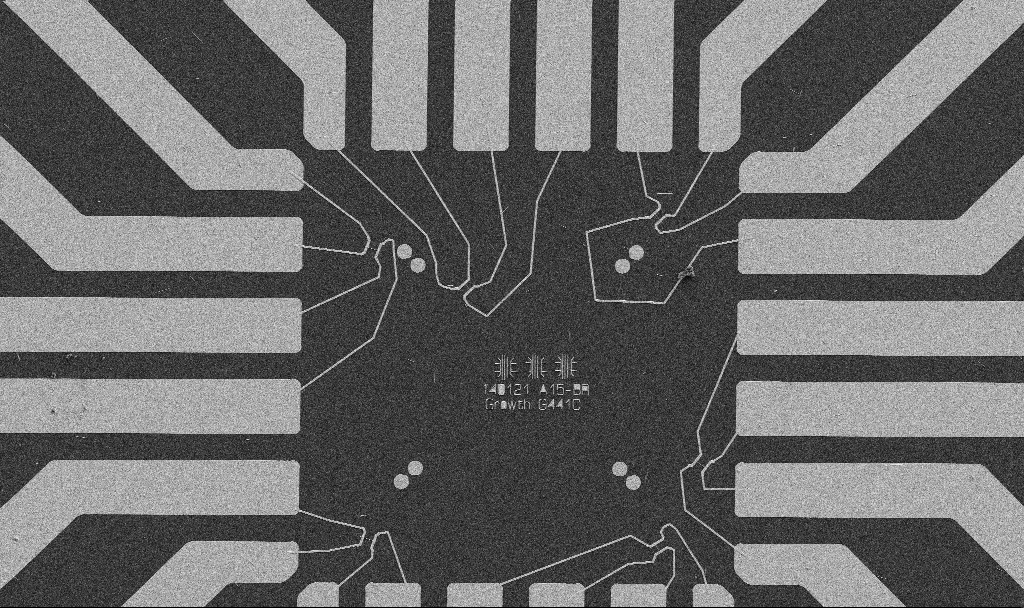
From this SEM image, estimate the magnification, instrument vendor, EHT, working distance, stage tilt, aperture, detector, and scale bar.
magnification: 1 K X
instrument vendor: Zeiss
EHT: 5 kV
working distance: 10.7 mm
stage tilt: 0°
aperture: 30 µm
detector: SE2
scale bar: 20000 nm